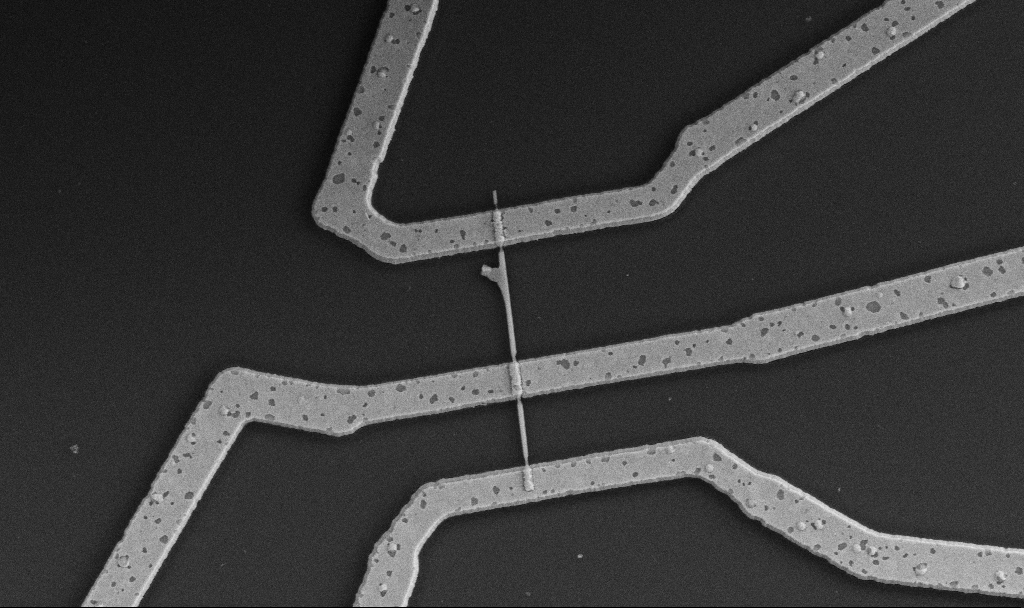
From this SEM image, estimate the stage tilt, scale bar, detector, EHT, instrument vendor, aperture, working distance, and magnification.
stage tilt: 0°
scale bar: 1000 nm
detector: SE2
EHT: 5 kV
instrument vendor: Zeiss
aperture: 30 µm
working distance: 10.6 mm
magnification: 20 K X